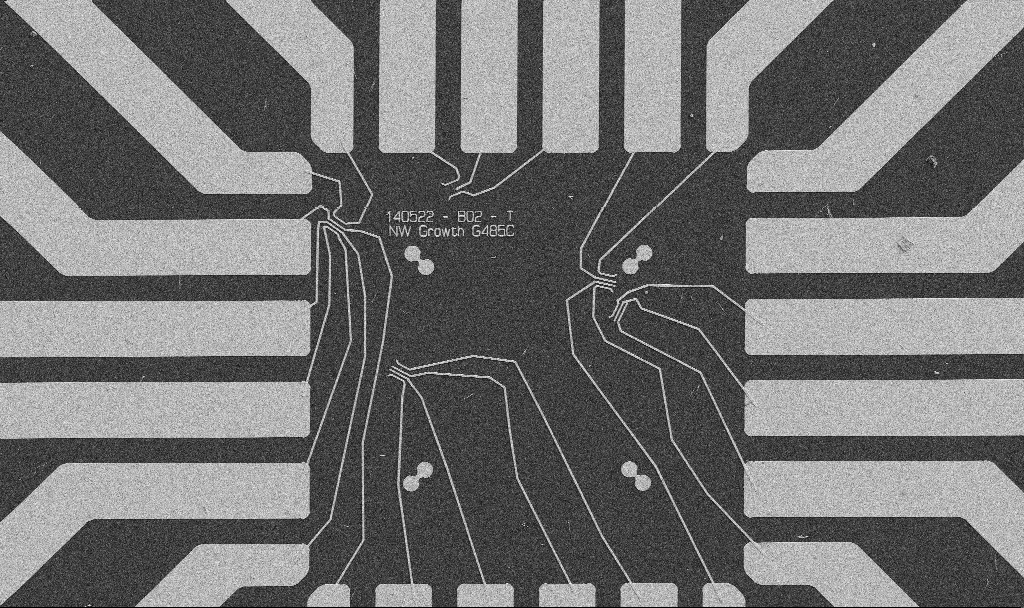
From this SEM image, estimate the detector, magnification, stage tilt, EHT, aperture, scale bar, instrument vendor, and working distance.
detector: SE2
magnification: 1 K X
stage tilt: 0°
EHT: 5 kV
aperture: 30 µm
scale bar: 20000 nm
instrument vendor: Zeiss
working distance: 10.7 mm